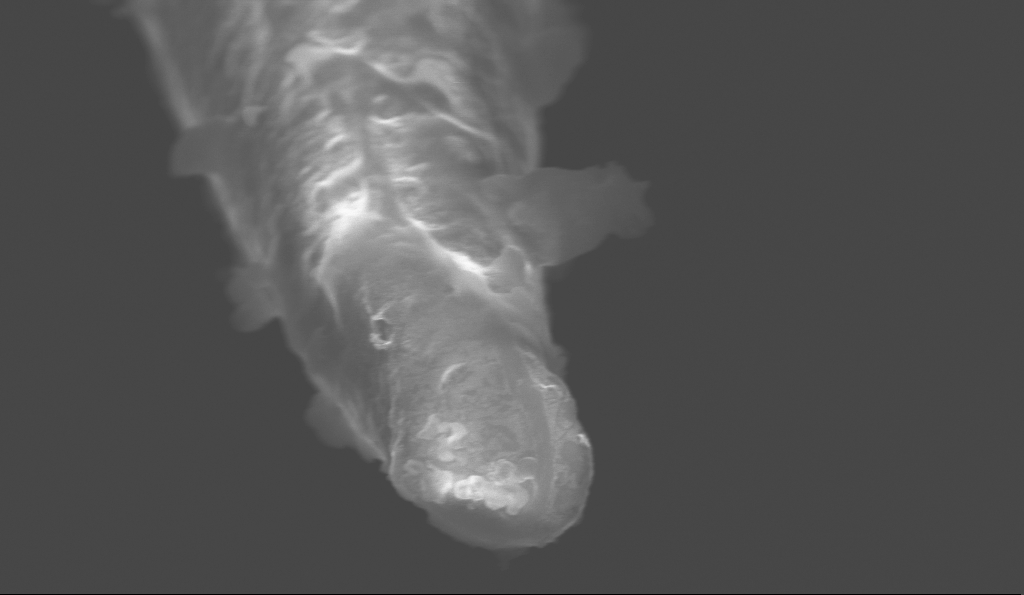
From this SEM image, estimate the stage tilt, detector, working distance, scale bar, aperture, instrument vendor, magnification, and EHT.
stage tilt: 70°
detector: InLens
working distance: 5.1 mm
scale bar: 200 nm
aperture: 30 µm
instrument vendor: Zeiss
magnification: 79.83 K X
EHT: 10 kV